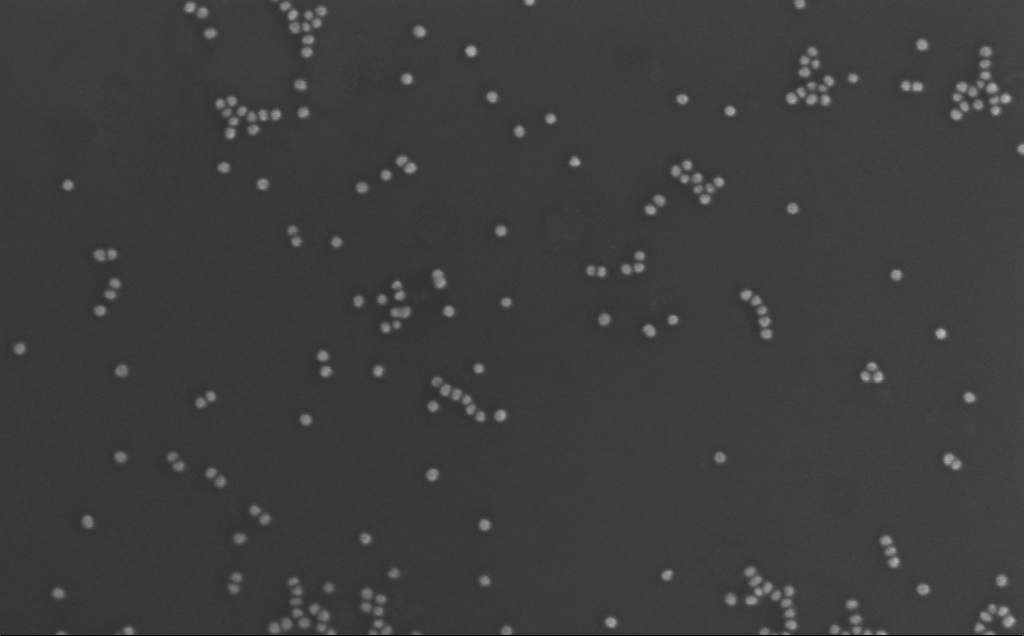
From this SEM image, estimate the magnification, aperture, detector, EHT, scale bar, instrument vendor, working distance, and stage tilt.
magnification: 234.19 K X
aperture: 30 µm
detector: InLens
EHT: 10 kV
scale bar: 200 nm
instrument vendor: Zeiss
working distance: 3 mm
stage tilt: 0°